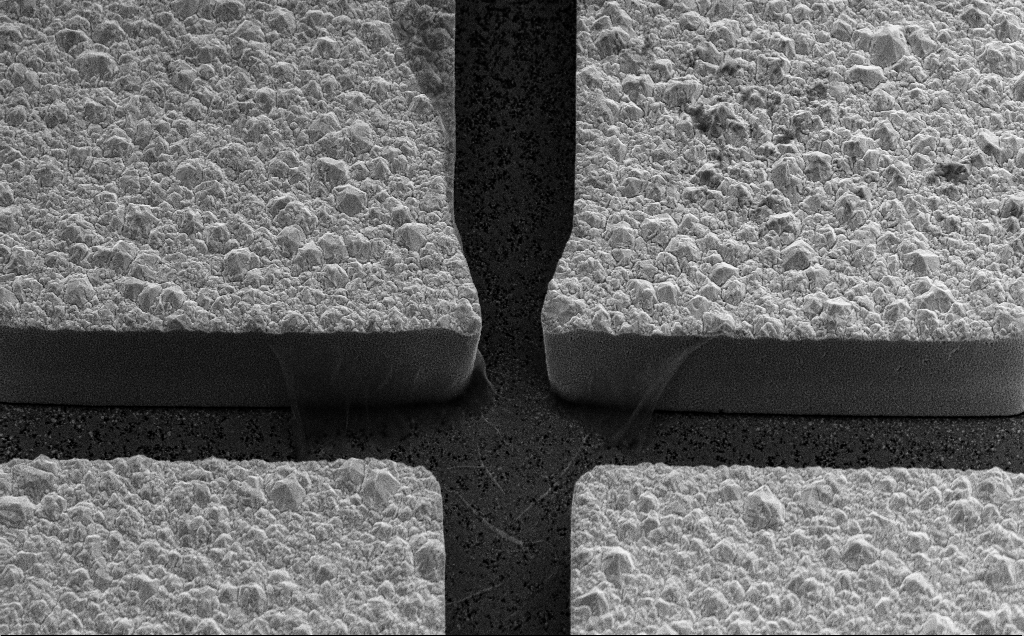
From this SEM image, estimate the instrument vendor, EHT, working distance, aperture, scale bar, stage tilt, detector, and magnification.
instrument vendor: Zeiss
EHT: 10 kV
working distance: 17 mm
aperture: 30 µm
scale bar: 10000 nm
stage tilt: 45°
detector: SE2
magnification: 4.85 K X